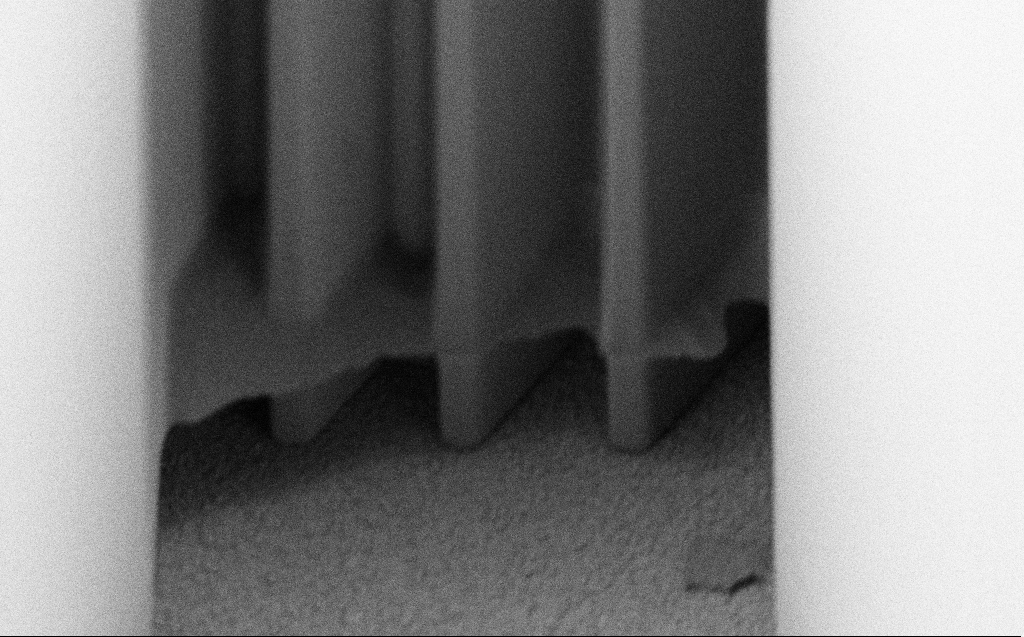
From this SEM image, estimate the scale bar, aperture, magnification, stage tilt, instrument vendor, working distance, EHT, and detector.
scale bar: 10000 nm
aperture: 30 µm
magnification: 5.51 K X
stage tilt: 45°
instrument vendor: Zeiss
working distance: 6 mm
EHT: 1 kV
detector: SE2